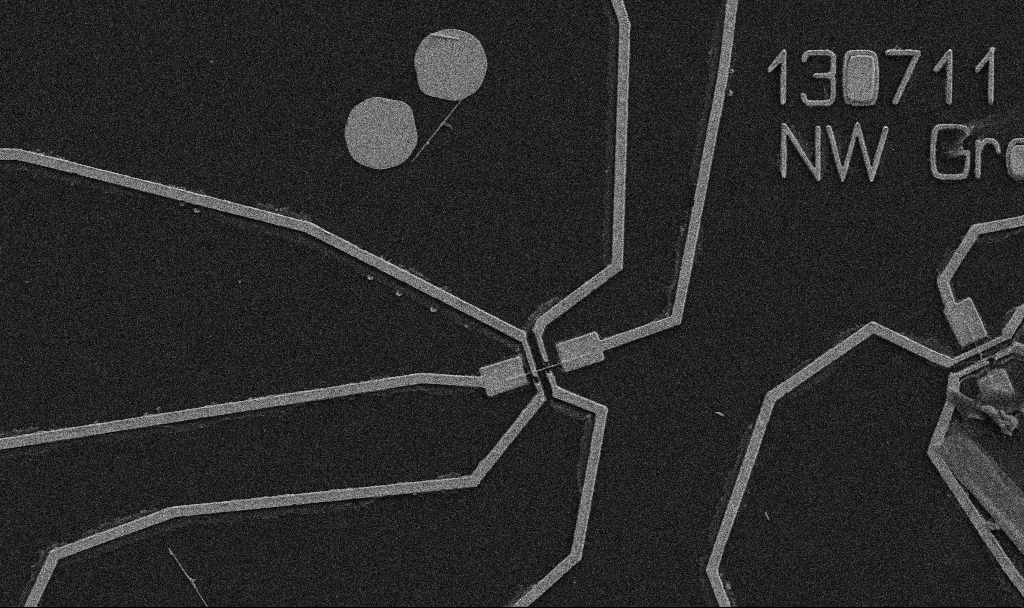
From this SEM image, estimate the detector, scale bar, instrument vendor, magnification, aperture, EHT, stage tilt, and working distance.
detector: SE2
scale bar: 10000 nm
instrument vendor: Zeiss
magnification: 5 K X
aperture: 30 µm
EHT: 5 kV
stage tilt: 0°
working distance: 10.7 mm